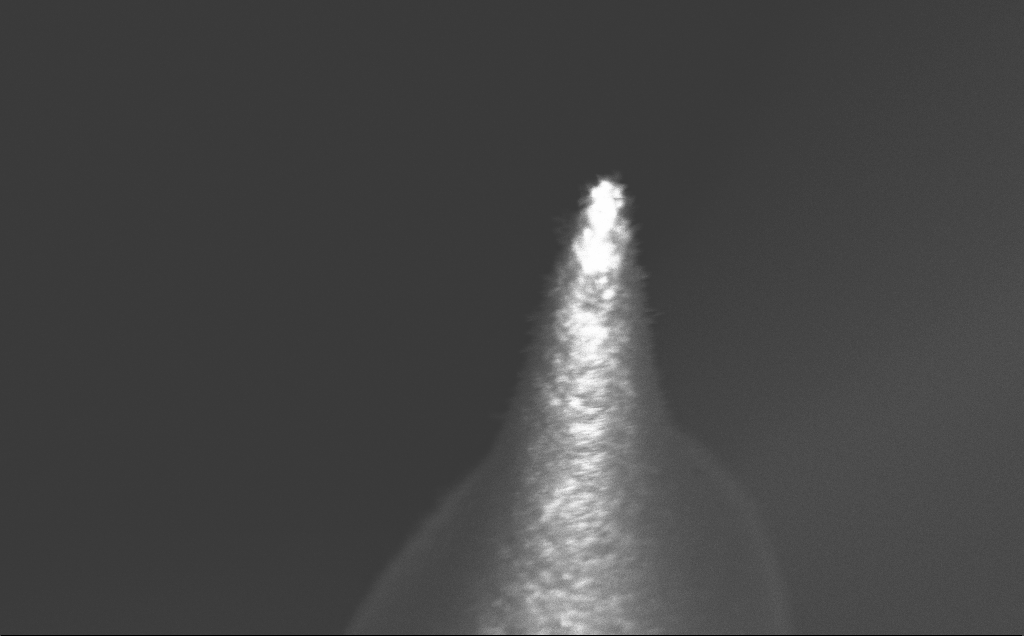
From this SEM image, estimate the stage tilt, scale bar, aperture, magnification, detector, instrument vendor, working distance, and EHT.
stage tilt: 20°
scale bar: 20000 nm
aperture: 30 µm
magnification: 3.17 K X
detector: InLens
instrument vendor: Zeiss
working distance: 5 mm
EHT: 5 kV